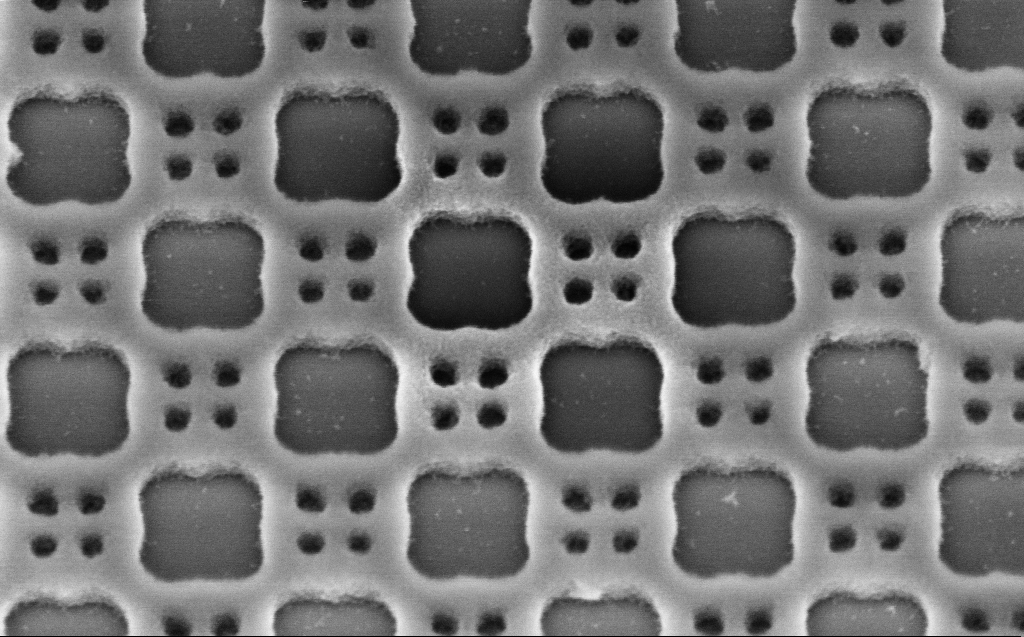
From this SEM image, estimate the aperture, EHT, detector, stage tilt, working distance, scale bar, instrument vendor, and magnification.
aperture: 30 µm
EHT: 3 kV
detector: InLens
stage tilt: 30°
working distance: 5 mm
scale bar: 200 nm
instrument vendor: Zeiss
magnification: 98.36 K X